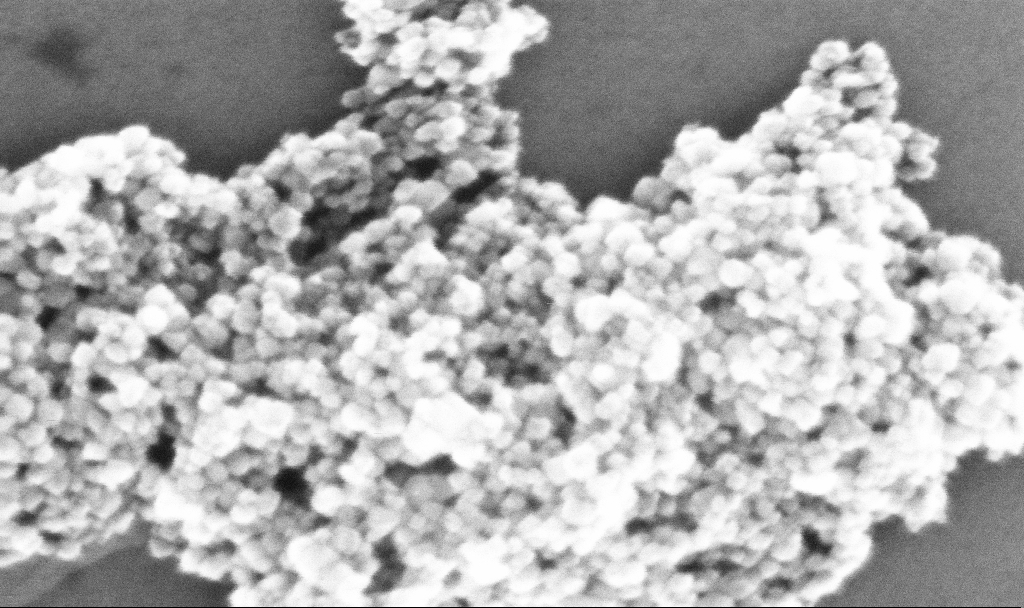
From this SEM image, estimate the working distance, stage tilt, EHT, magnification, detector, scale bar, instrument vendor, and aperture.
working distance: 5.2 mm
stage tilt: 0°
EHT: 10 kV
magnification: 454.64 K X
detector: InLens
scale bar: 100 nm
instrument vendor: Zeiss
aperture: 30 µm